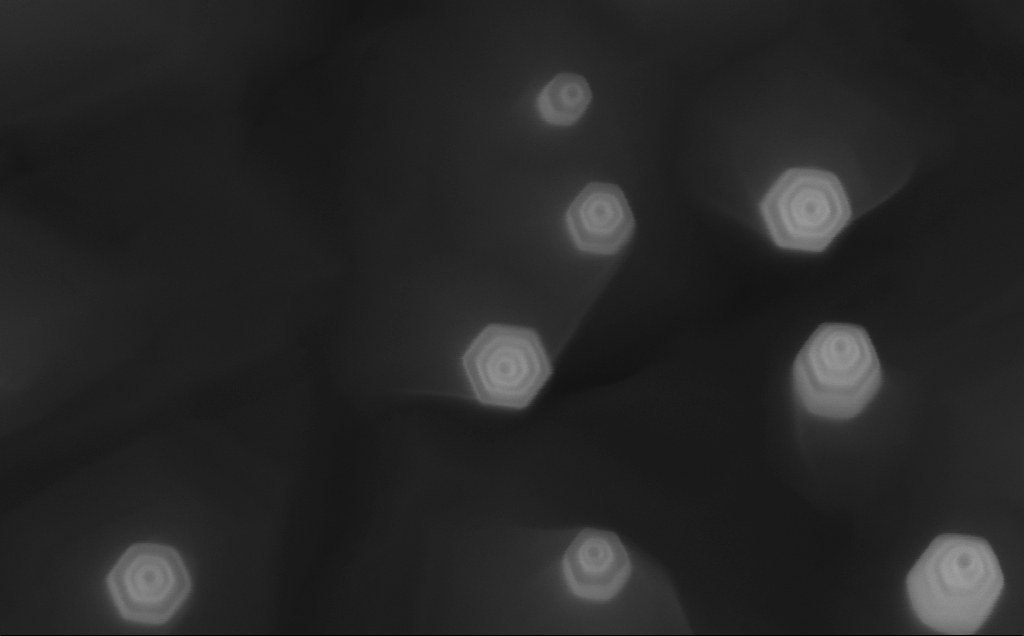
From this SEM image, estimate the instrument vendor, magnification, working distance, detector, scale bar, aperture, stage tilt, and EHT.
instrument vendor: Zeiss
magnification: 300 K X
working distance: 5 mm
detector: InLens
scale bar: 200 nm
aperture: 30 µm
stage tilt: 0°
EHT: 10 kV